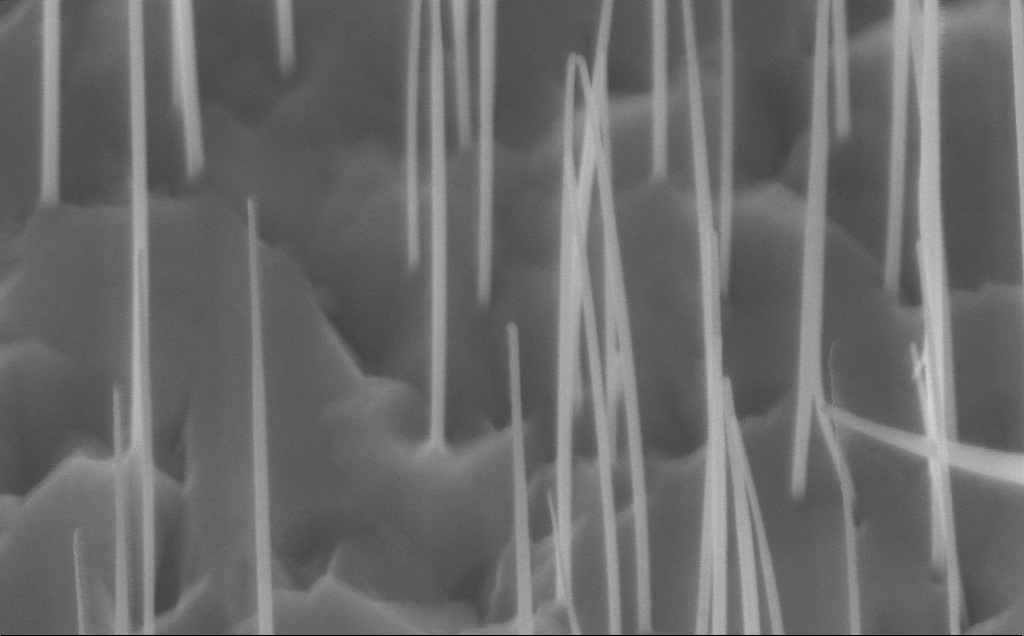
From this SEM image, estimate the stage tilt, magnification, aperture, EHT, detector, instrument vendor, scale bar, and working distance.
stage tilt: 45°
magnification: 150 K X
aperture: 30 µm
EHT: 10 kV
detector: InLens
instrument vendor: Zeiss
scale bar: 200 nm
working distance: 7 mm